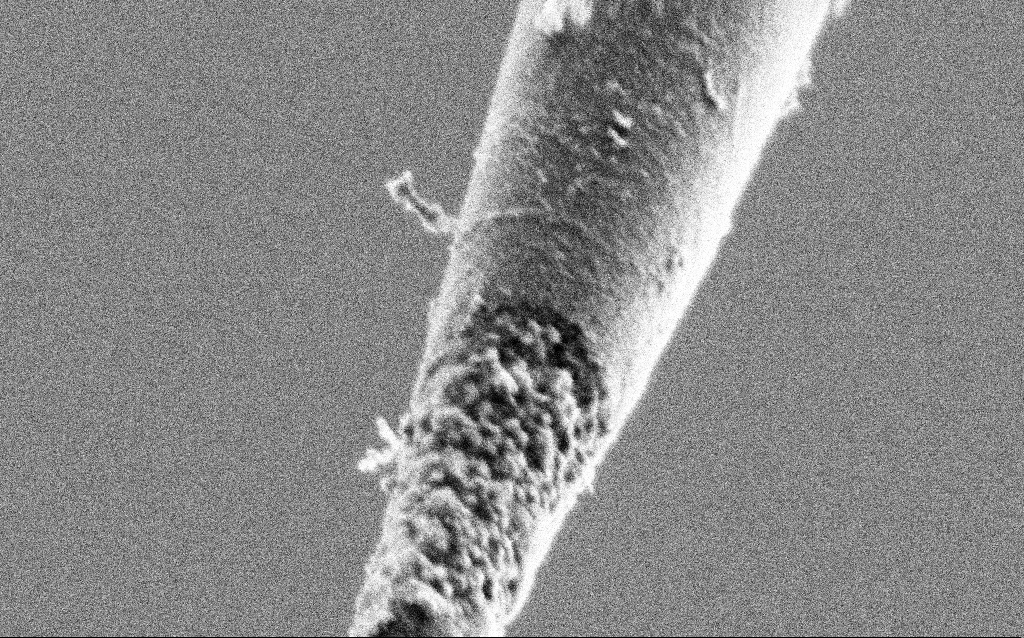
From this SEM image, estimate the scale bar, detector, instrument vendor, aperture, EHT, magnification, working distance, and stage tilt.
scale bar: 200 nm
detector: SE2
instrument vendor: Zeiss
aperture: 30 µm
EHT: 1 kV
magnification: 100 K X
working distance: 6.5 mm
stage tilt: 45°